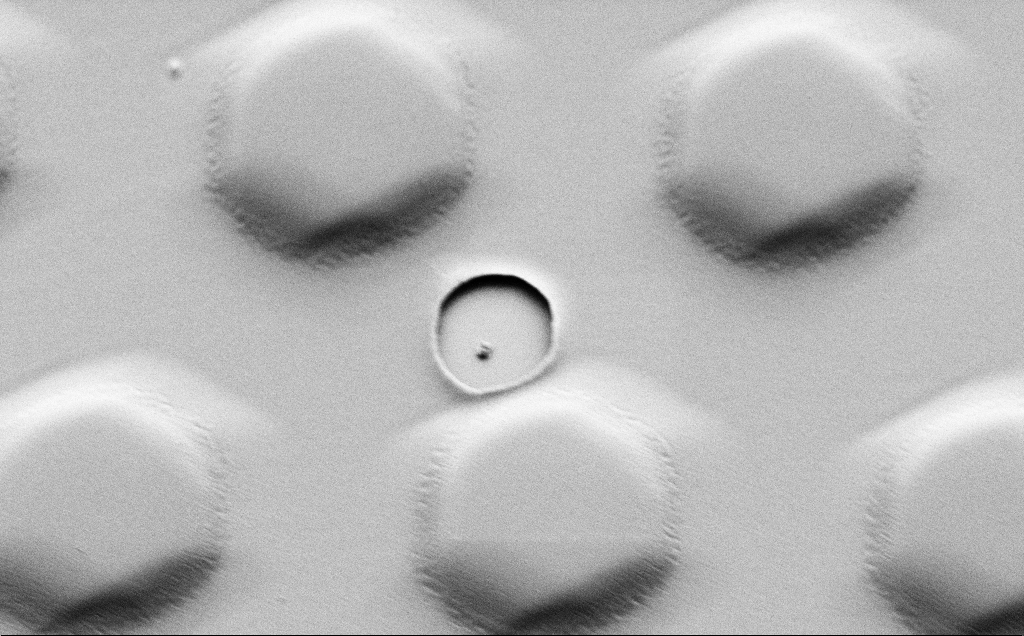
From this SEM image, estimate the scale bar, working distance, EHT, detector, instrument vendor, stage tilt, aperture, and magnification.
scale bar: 2000 nm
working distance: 4 mm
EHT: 1.5 kV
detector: SE2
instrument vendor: Zeiss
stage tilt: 45°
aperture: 30 µm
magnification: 8.43 K X